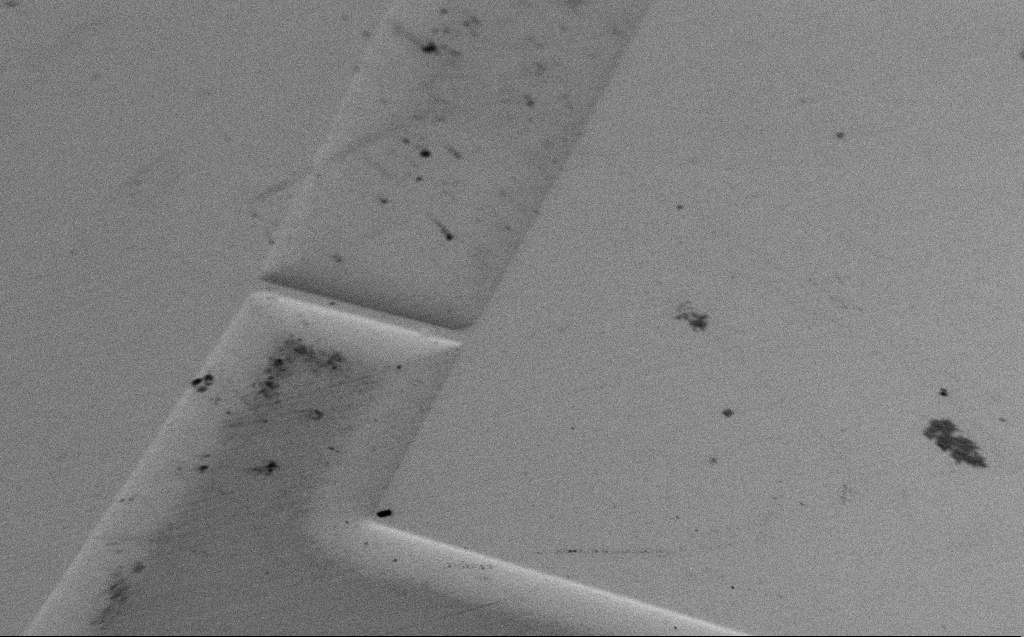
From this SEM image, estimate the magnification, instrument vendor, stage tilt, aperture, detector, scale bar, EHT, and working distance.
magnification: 0.635 K X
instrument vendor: Zeiss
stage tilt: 45°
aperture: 30 µm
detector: SE2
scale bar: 100000 nm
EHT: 1.2 kV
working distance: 7 mm